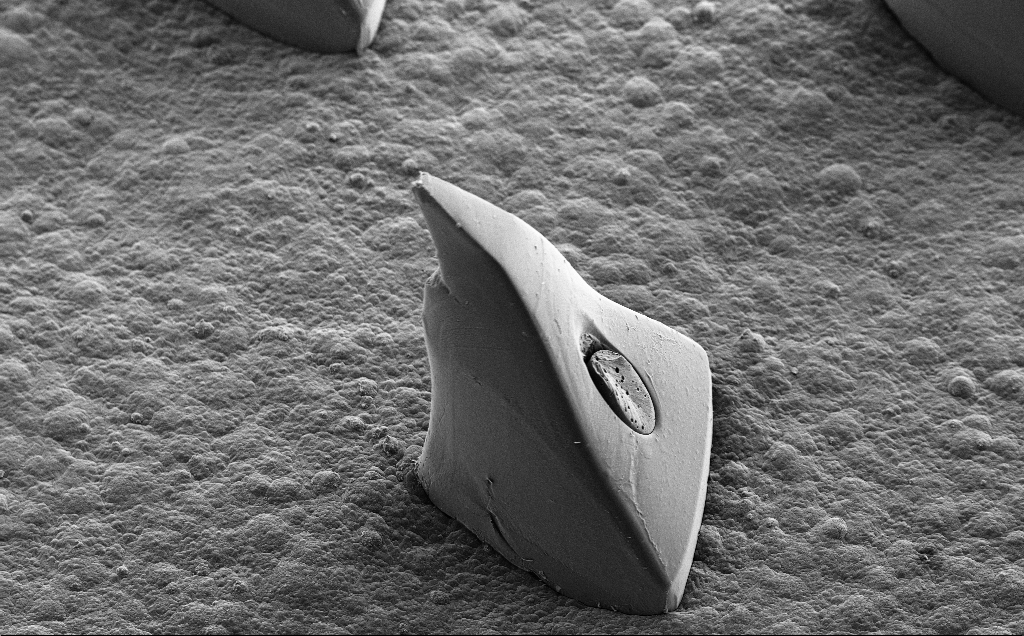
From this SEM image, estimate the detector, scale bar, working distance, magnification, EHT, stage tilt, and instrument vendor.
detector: SE2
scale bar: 200000 nm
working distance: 10 mm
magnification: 0.195 K X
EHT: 10 kV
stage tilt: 35.3°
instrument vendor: Zeiss